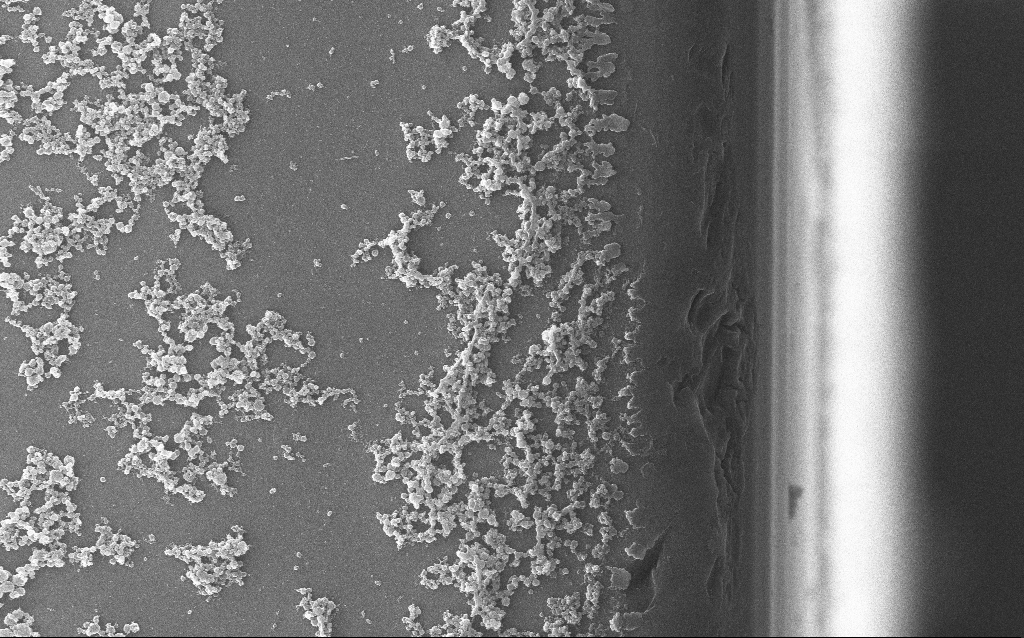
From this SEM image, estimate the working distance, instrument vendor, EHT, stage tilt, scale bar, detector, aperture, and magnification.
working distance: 1.7 mm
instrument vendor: Zeiss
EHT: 20 kV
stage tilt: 0°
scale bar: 2000 nm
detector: InLens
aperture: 30 µm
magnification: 10 K X